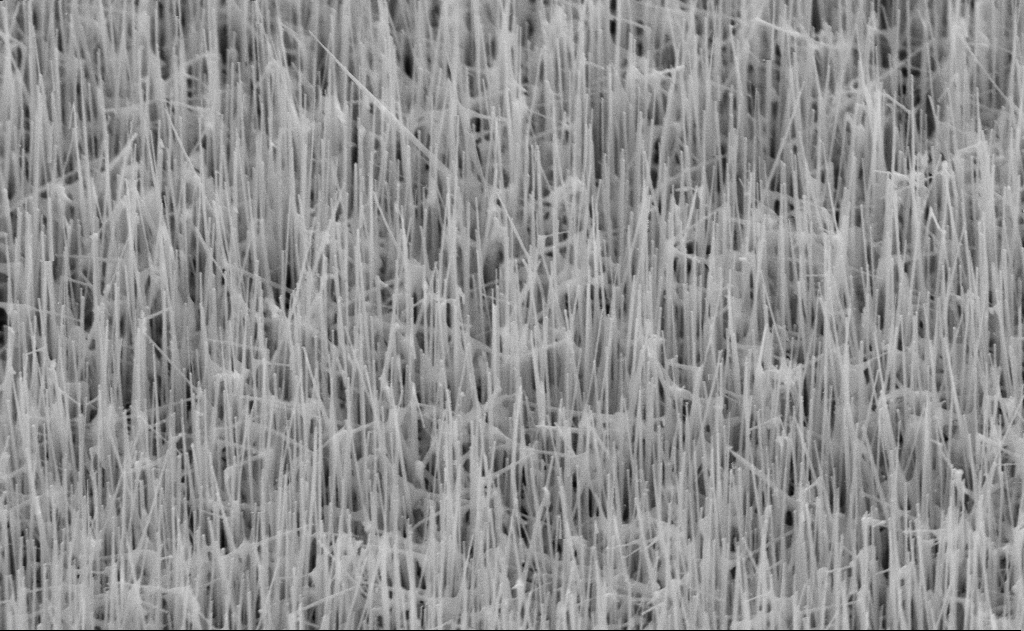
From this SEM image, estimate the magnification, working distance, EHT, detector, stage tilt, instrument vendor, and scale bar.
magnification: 40 K X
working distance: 11 mm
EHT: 10 kV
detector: SE2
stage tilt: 45°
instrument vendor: Zeiss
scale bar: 1000 nm